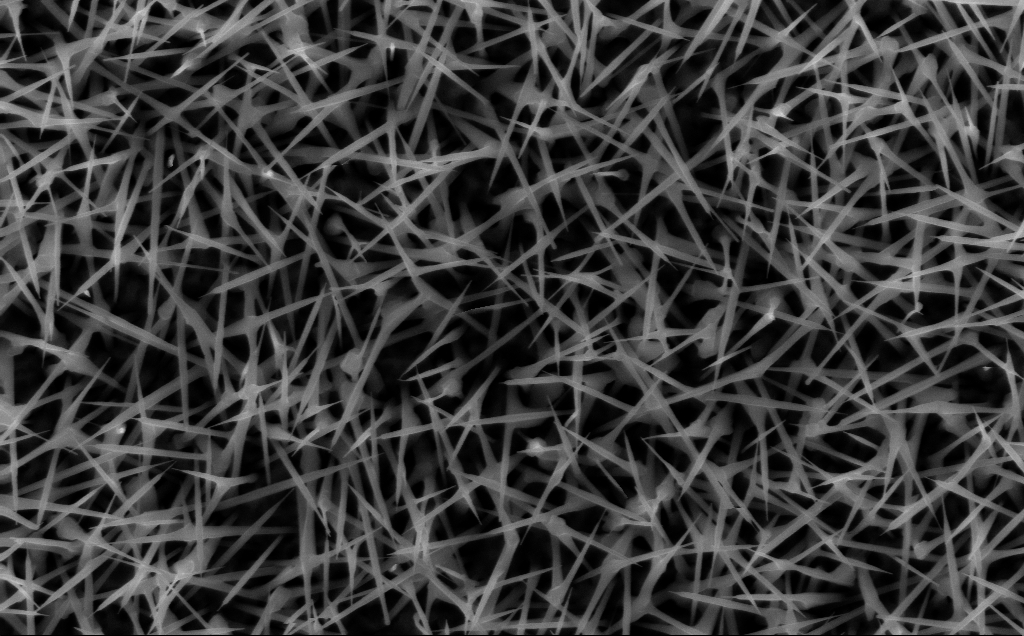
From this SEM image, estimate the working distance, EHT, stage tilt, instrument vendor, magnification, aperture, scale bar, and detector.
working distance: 7 mm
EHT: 10 kV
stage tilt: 0°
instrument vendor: Zeiss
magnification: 40 K X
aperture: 30 µm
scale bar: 1000 nm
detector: InLens